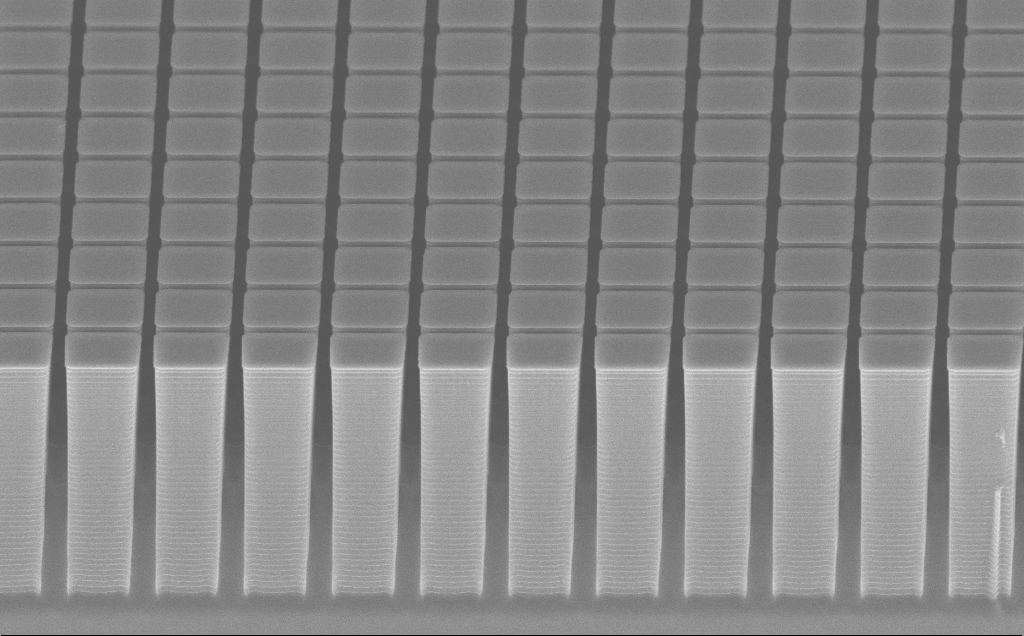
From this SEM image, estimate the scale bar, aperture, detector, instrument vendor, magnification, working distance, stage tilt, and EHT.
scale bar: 10000 nm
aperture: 30 µm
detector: InLens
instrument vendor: Zeiss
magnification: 6.54 K X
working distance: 8 mm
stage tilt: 60.9°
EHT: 10 kV